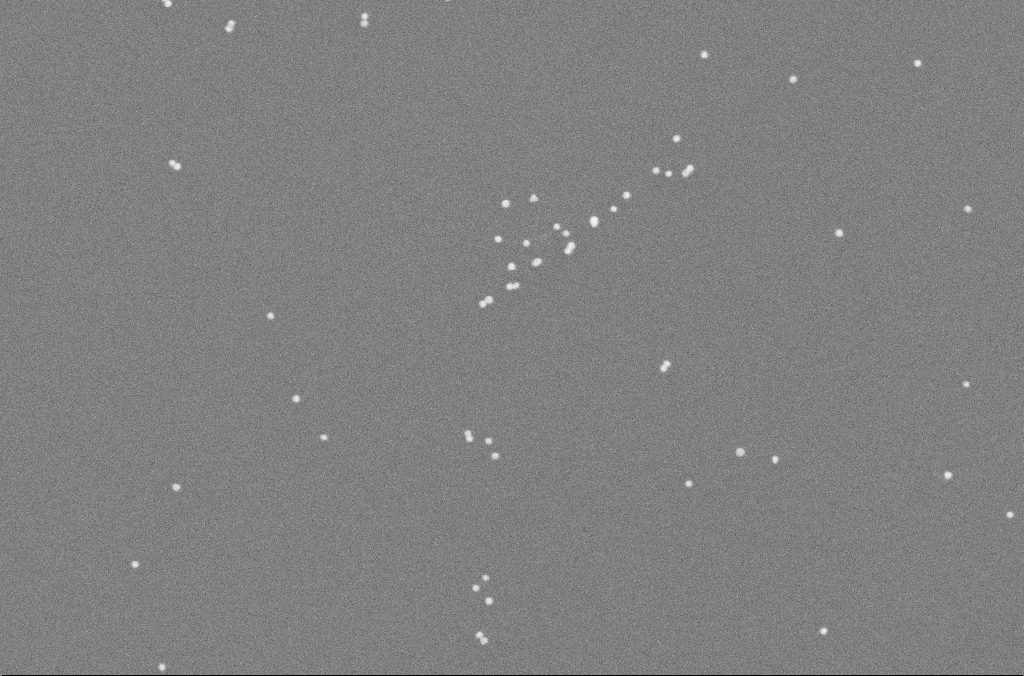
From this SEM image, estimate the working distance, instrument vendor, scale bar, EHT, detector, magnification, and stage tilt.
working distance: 2.5 mm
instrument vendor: Zeiss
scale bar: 200 nm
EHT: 10 kV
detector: SE2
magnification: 128.44 K X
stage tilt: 0°